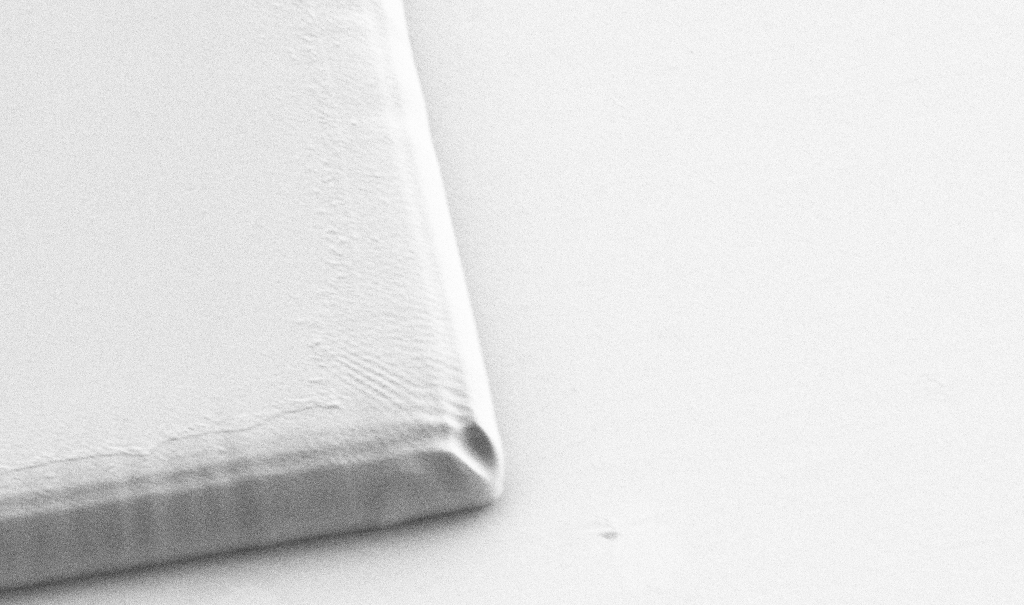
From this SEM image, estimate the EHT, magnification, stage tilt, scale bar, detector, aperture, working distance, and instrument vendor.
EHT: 1 kV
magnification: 6.45 K X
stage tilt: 36°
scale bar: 10000 nm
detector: SE2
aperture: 30 µm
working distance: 6 mm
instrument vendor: Zeiss